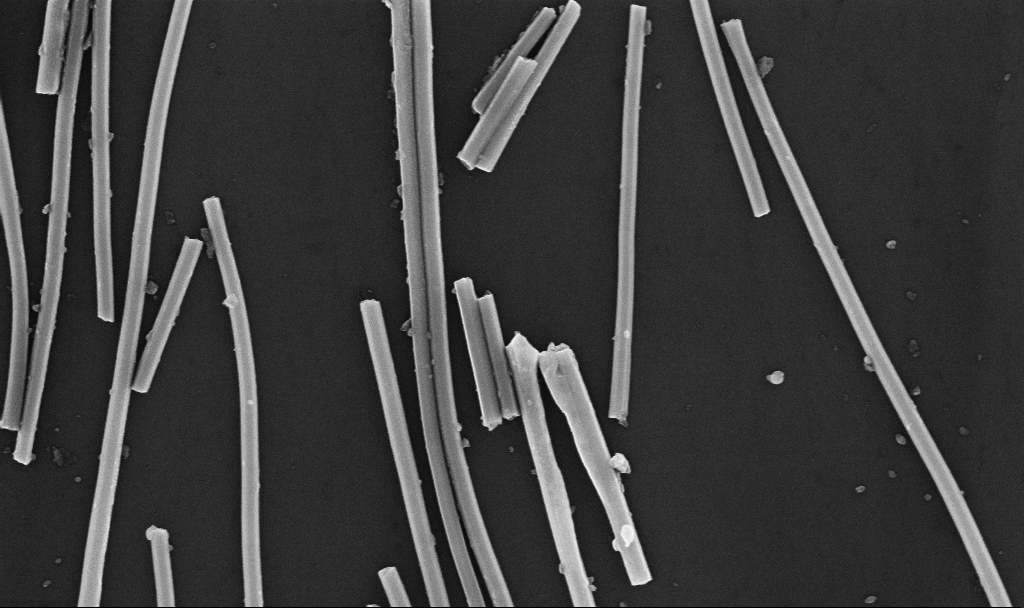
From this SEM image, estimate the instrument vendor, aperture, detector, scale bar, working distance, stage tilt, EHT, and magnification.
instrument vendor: Zeiss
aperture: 30 µm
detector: InLens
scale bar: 1000 nm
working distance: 6.7 mm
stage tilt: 0°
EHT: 10 kV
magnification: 62.85 K X